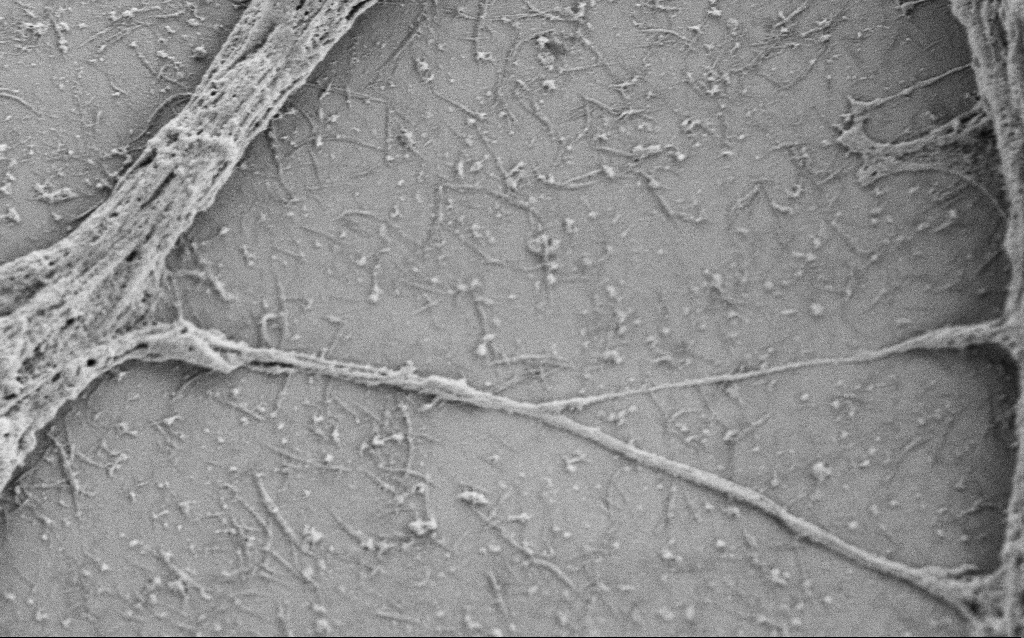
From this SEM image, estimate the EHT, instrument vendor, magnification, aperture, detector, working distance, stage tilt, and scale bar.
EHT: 0.8 kV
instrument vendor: Zeiss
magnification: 20 K X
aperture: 30 µm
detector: SE2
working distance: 5.7 mm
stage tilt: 0°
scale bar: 1000 nm